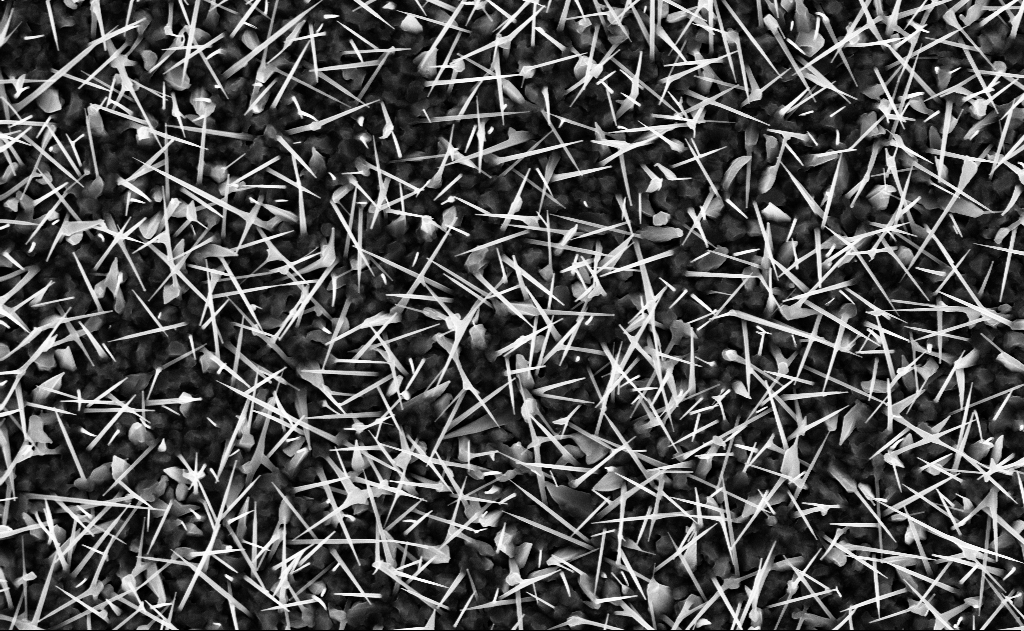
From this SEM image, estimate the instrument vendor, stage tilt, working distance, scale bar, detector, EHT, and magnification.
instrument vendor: Zeiss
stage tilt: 0°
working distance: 9 mm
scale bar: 2000 nm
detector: InLens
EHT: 10 kV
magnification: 20 K X